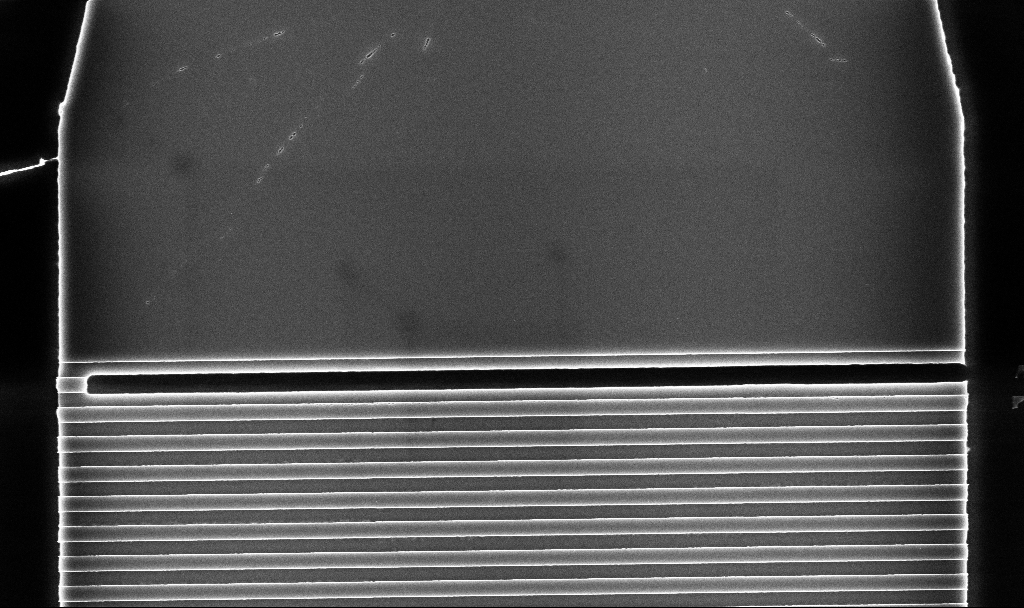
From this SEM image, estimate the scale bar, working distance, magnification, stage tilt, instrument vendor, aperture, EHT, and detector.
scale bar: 2000 nm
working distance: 5.2 mm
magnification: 16.84 K X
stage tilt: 0°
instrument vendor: Zeiss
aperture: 30 µm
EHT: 5 kV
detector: InLens